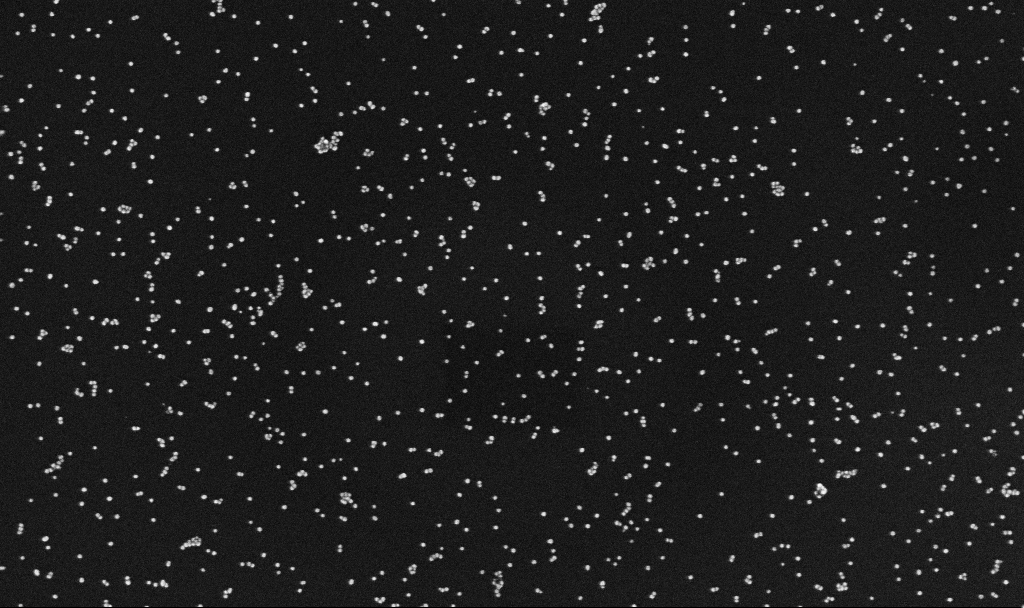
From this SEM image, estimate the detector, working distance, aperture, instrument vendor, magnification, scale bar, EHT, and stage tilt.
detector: InLens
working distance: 3.4 mm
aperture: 30 µm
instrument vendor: Zeiss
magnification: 100 K X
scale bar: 200 nm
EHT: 10 kV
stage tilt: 0°